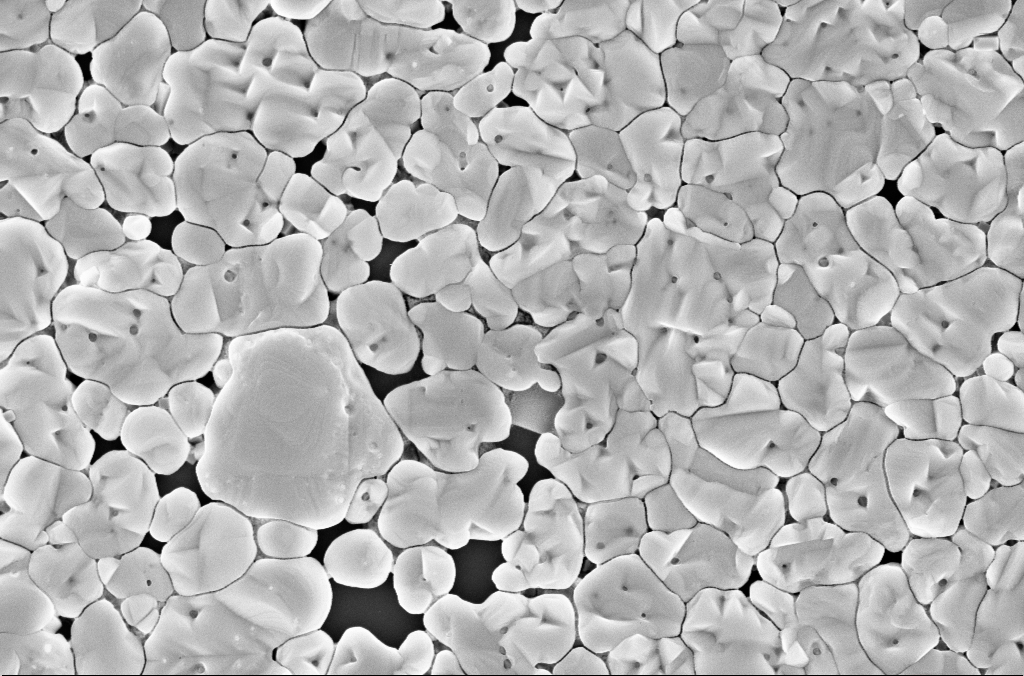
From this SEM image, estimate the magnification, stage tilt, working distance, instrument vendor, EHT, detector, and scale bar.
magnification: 40 K X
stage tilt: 0°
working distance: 3 mm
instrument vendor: Zeiss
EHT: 5 kV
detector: InLens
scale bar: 1000 nm